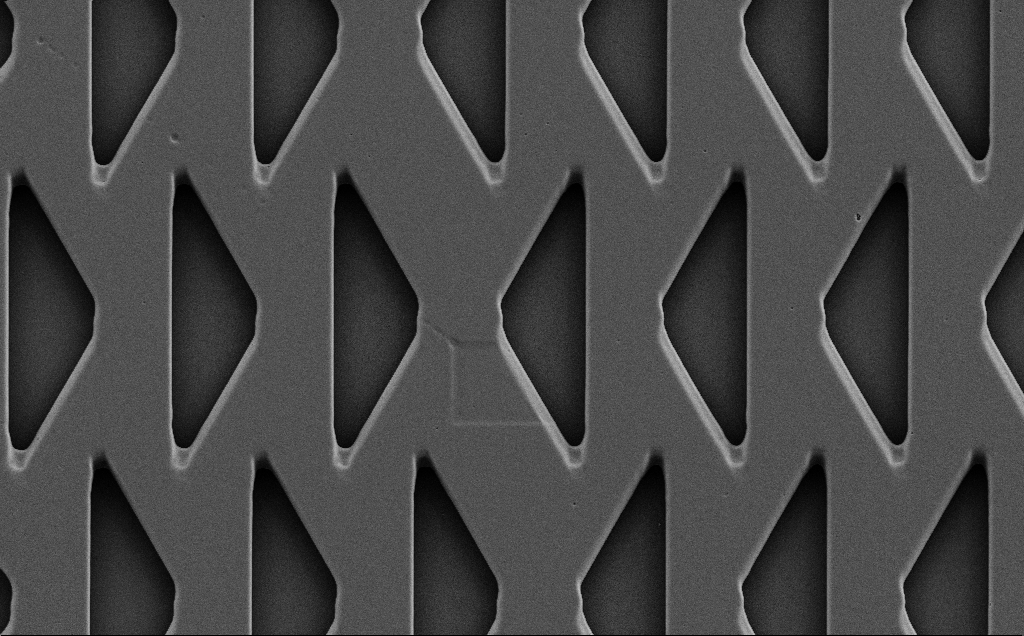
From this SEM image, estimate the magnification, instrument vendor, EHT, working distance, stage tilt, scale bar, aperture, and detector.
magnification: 2.47 K X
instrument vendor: Zeiss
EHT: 5 kV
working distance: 9 mm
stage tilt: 0°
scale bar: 10000 nm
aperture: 30 µm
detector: SE2